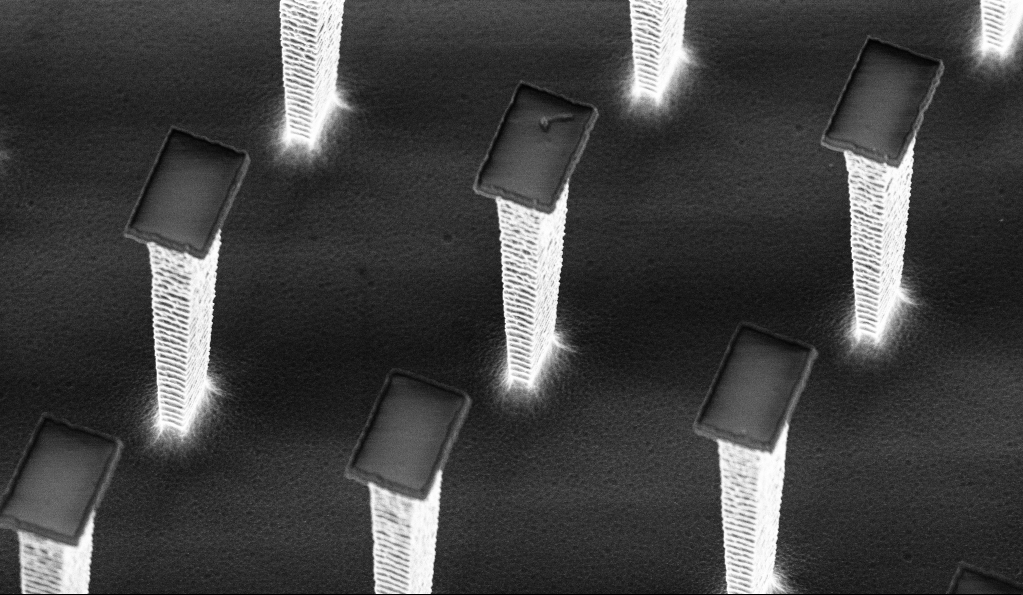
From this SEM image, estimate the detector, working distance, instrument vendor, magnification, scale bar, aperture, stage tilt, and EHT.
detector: InLens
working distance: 5.7 mm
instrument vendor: Zeiss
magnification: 10.73 K X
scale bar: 2000 nm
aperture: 30 µm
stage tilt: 30°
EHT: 3 kV